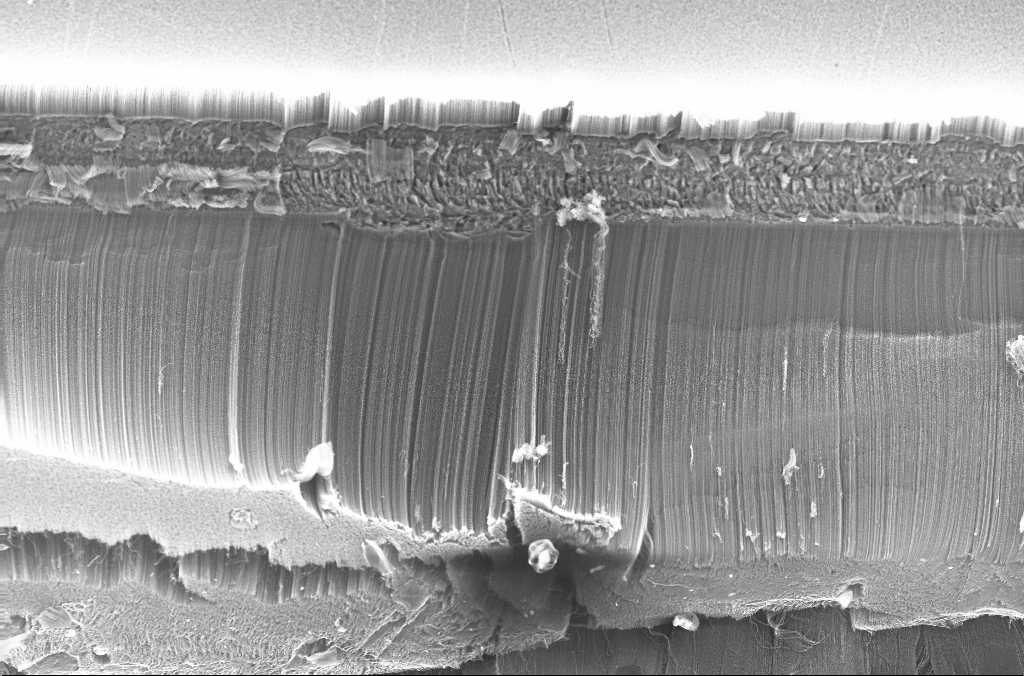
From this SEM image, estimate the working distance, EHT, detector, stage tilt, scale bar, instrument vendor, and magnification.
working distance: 4.2 mm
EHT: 20 kV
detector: InLens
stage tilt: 0°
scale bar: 100000 nm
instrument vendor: Zeiss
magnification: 0.5 K X